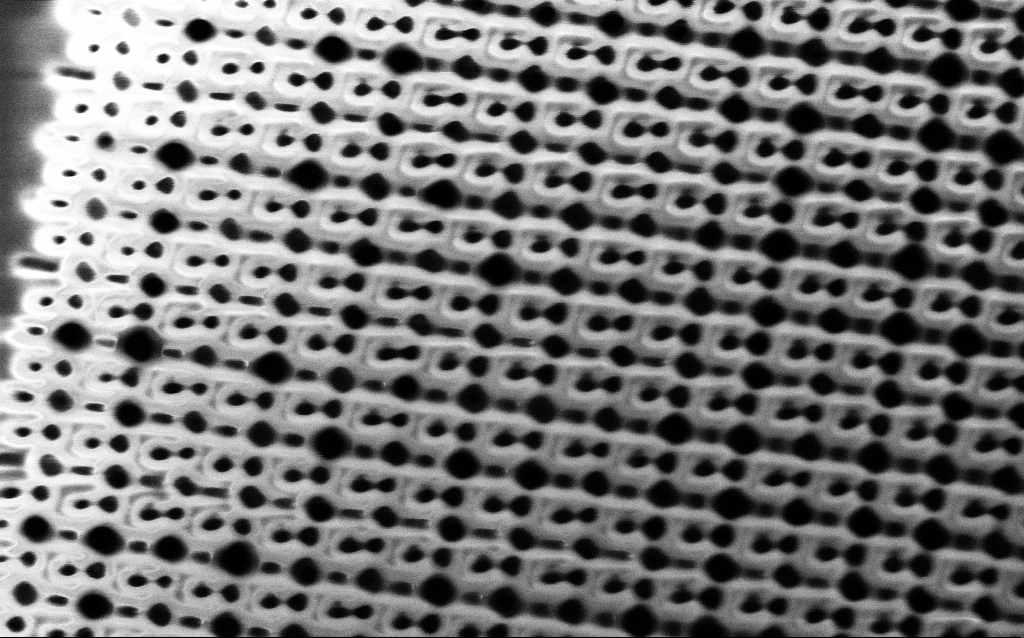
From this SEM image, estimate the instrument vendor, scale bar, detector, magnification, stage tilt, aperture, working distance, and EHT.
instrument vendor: Zeiss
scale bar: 1000 nm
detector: SE2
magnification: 53.04 K X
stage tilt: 0°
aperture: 30 µm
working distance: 6.2 mm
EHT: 1.5 kV